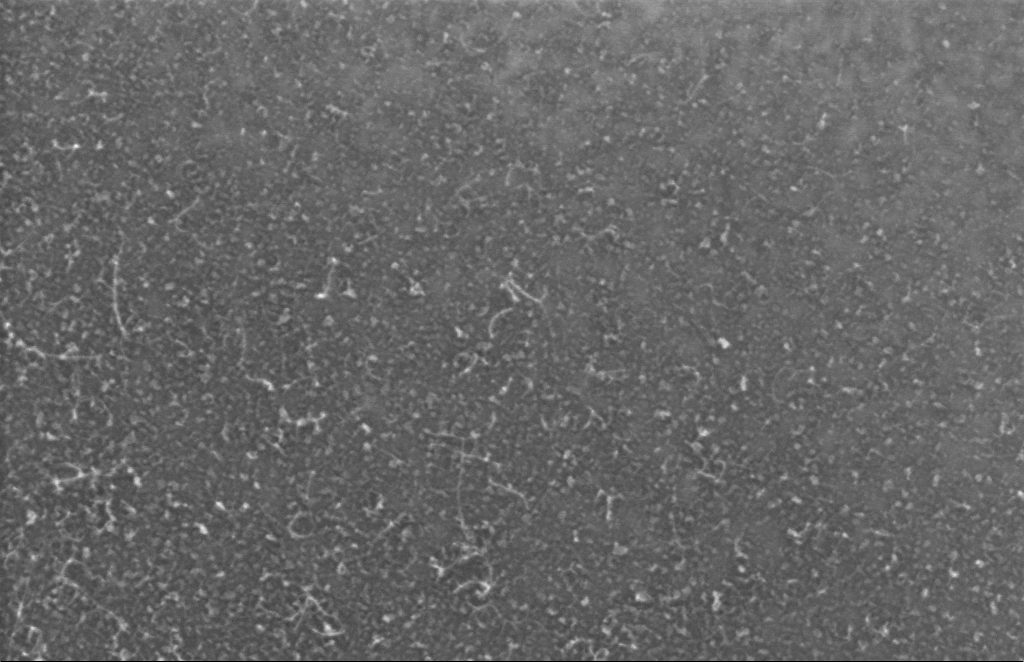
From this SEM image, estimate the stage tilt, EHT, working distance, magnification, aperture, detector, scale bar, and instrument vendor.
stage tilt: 0°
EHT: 5 kV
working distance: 6 mm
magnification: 280.36 K X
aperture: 30 µm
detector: InLens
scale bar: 100 nm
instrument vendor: Zeiss